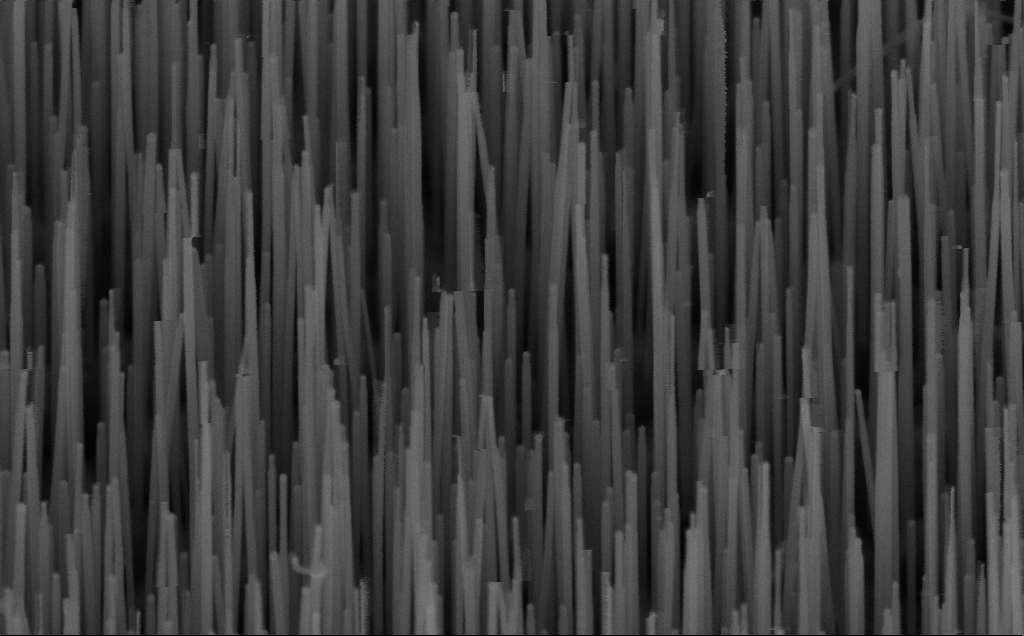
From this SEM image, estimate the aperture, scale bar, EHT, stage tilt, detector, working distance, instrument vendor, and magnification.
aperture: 30 µm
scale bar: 200 nm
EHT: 10 kV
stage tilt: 45°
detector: InLens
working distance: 7 mm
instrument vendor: Zeiss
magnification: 100 K X